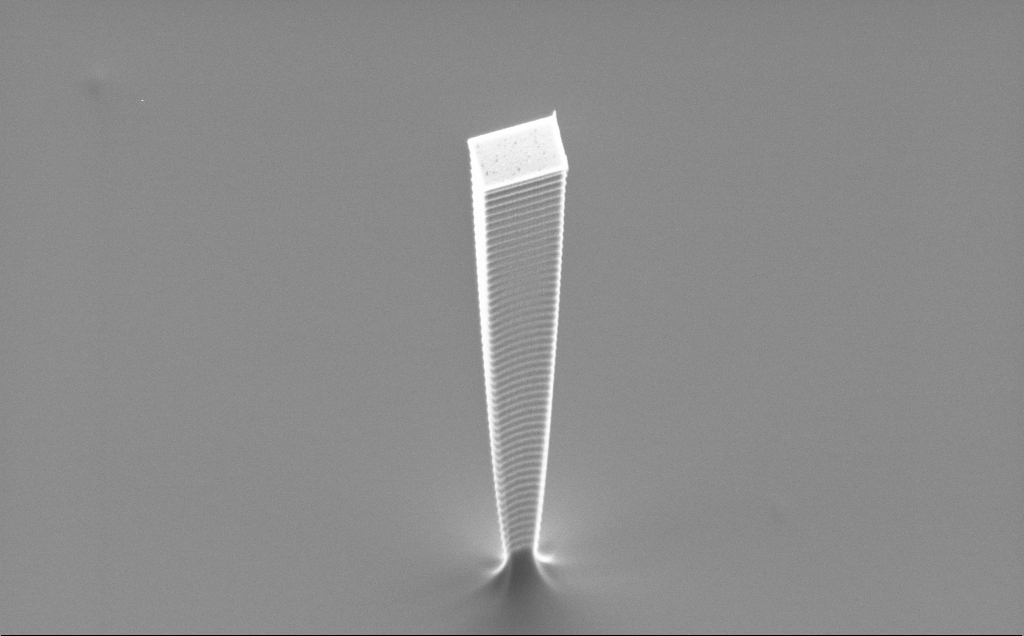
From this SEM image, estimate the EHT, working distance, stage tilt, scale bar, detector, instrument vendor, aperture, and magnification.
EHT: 5 kV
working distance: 16 mm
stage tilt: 50°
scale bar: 10000 nm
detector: SE2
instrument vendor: Zeiss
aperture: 30 µm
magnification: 6.72 K X